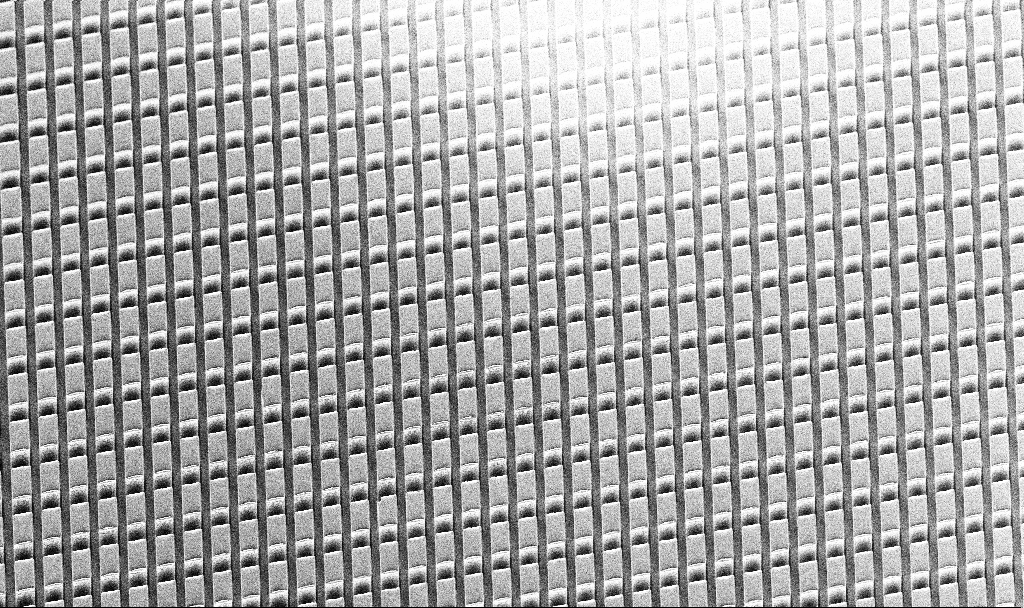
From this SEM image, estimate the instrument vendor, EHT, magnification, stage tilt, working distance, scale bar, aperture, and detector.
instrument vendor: Zeiss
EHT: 5 kV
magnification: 0.849 K X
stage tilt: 30°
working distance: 9.3 mm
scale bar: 20000 nm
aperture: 30 µm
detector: InLens